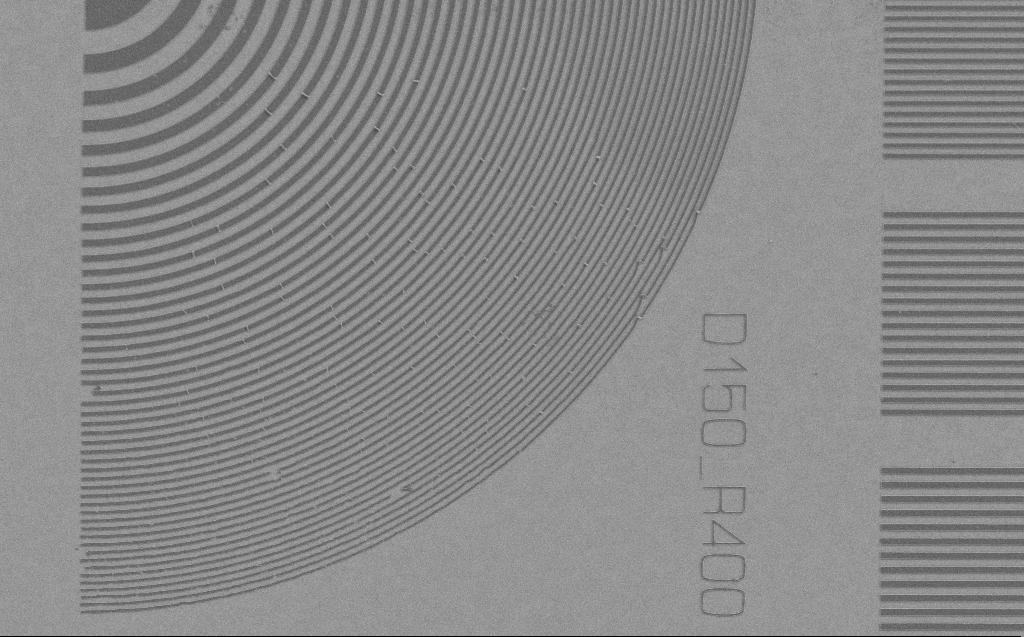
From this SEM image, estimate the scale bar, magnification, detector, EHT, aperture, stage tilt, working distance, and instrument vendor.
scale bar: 10000 nm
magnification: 3.28 K X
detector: SE2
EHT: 1.2 kV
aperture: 30 µm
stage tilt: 0°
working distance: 5 mm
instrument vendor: Zeiss